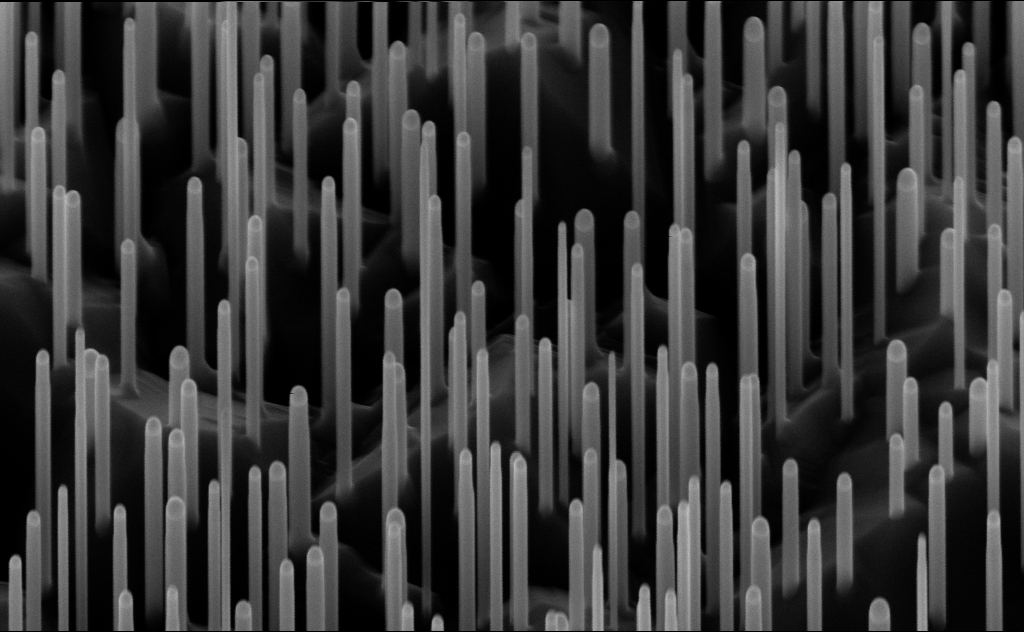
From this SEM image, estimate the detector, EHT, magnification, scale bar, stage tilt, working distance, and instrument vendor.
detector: InLens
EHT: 10 kV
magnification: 80 K X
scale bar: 200 nm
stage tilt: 45°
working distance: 7 mm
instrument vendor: Zeiss